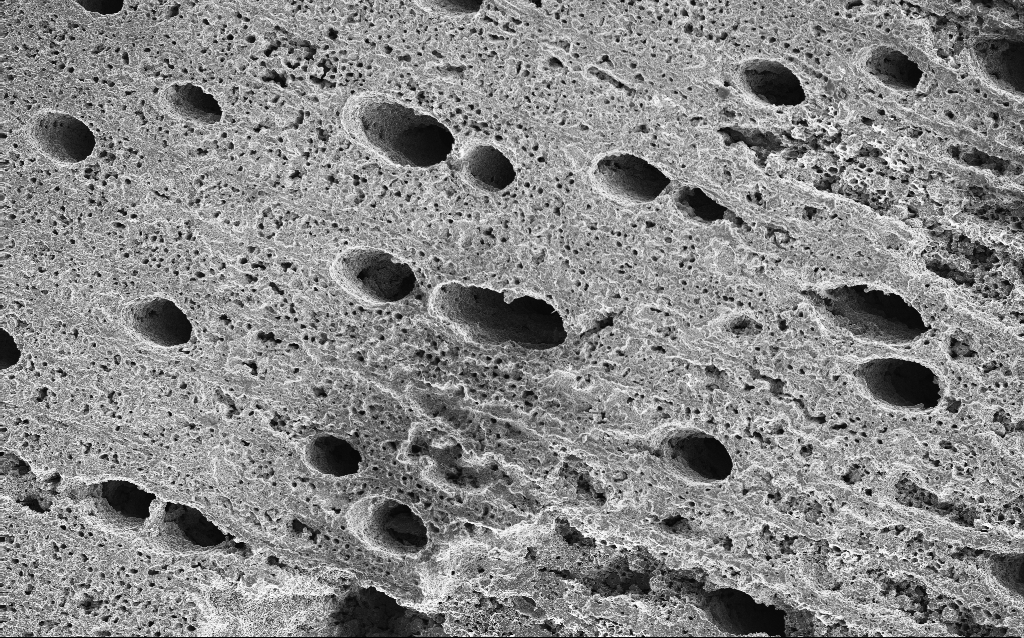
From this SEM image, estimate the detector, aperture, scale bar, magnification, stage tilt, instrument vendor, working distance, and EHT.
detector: InLens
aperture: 30 µm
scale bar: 100000 nm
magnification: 0.189 K X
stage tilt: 0°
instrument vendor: Zeiss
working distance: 3 mm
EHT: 10 kV